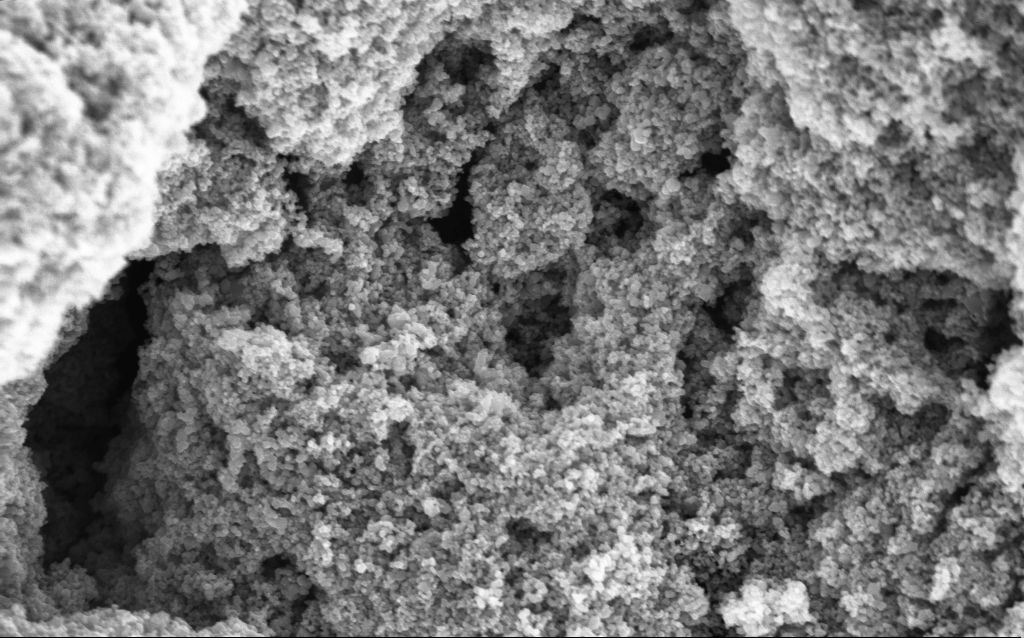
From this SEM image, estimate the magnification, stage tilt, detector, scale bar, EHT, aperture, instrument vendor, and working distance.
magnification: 68.65 K X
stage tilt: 0°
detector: InLens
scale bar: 1000 nm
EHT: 5 kV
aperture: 30 µm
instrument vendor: Zeiss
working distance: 4.7 mm